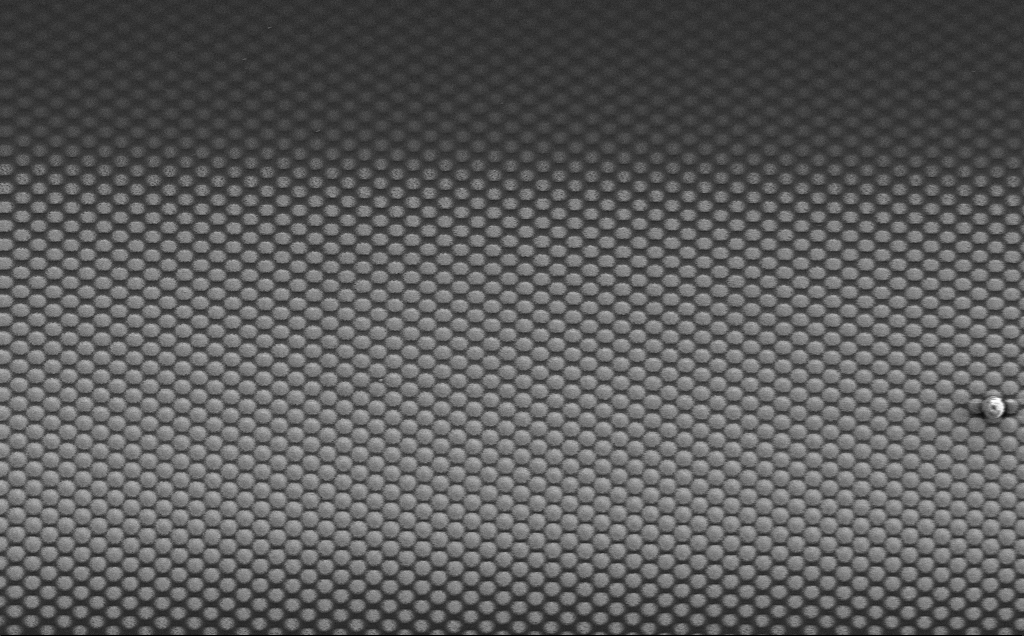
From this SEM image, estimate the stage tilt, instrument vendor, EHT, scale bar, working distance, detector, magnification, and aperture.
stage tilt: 0°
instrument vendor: Zeiss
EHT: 5 kV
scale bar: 20000 nm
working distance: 20 mm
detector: SE2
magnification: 2.27 K X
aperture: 30 µm